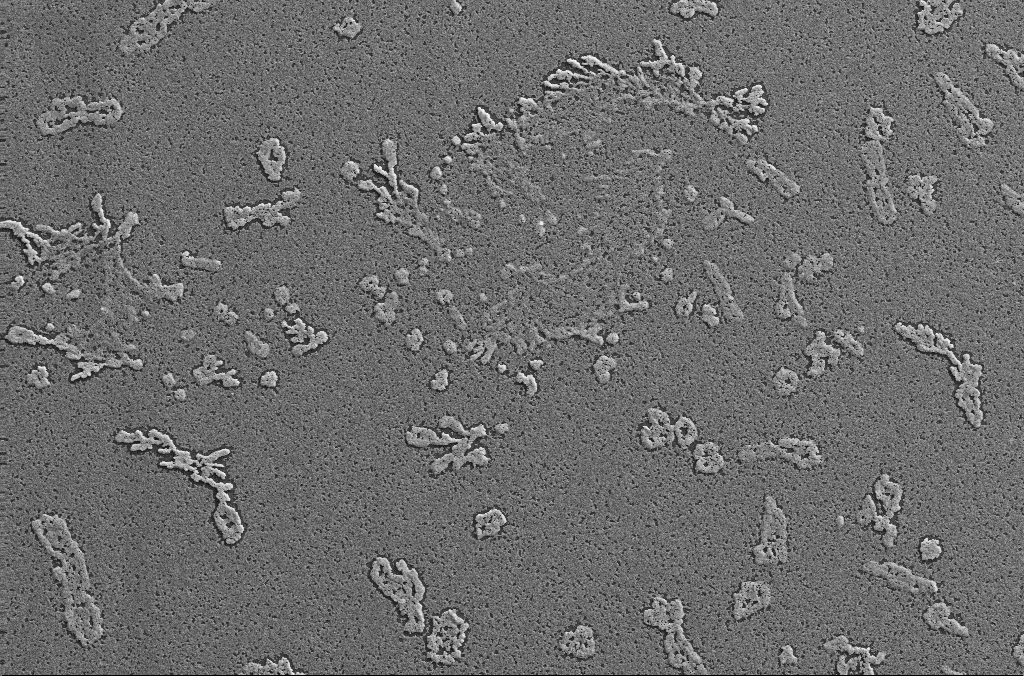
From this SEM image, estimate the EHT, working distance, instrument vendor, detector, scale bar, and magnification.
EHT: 20 kV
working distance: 2.9 mm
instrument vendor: Zeiss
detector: SE2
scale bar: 100000 nm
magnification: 0.5 K X